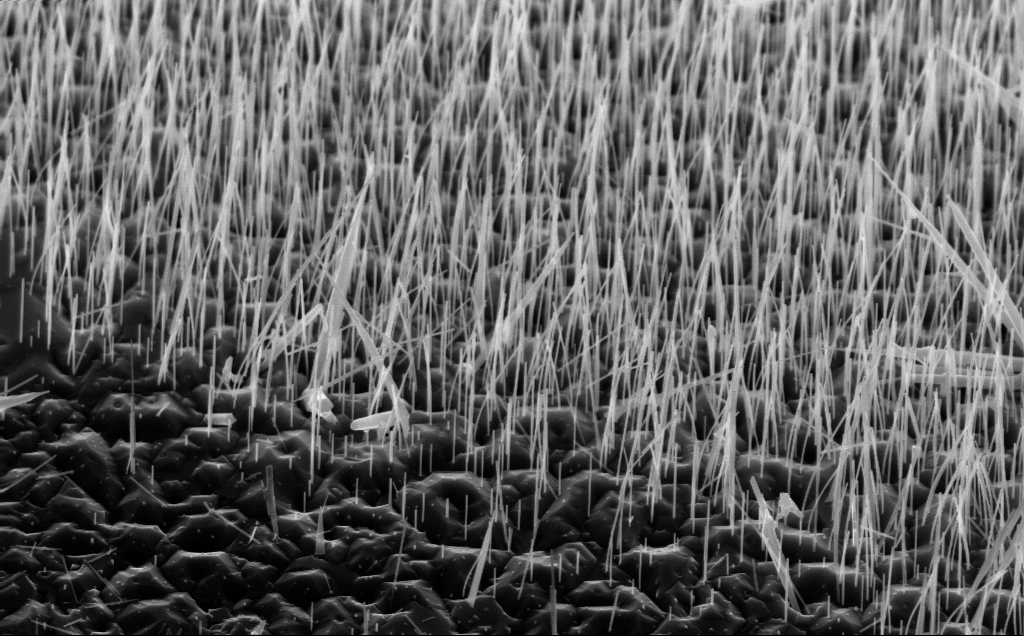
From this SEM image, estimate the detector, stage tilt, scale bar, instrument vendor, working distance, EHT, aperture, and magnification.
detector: InLens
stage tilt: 45°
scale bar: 2000 nm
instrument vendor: Zeiss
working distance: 5 mm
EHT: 10 kV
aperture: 30 µm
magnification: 21.58 K X